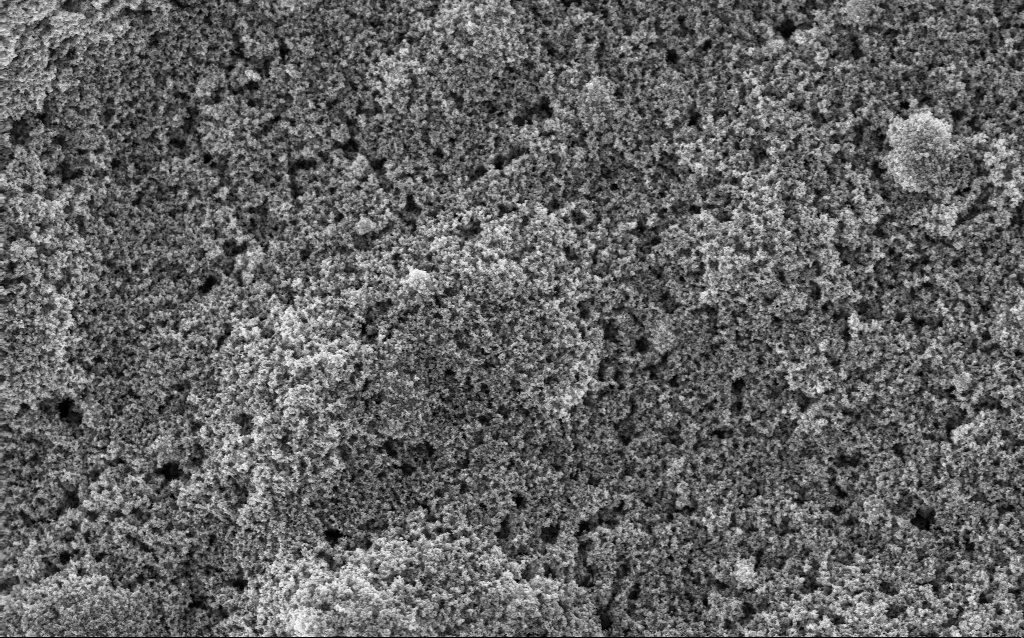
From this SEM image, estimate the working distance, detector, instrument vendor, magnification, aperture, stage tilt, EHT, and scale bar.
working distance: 2.9 mm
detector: InLens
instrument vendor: Zeiss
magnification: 23.9 K X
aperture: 30 µm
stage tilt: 0°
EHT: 5 kV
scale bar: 1000 nm